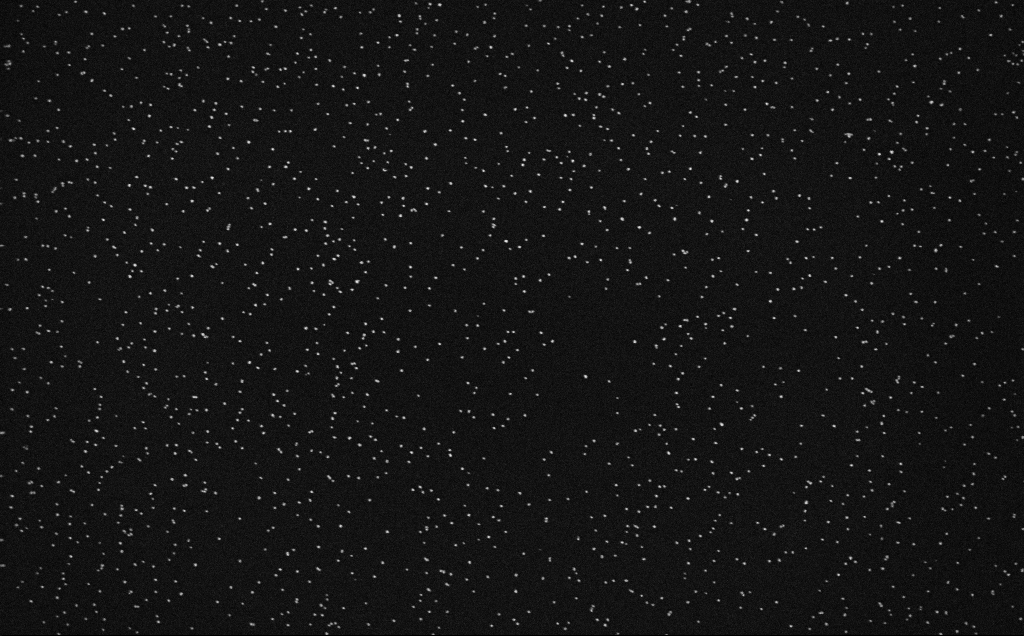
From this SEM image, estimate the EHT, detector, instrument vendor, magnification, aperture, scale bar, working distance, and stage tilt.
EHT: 10 kV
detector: InLens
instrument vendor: Zeiss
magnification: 100 K X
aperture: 30 µm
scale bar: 200 nm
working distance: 4 mm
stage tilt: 0°